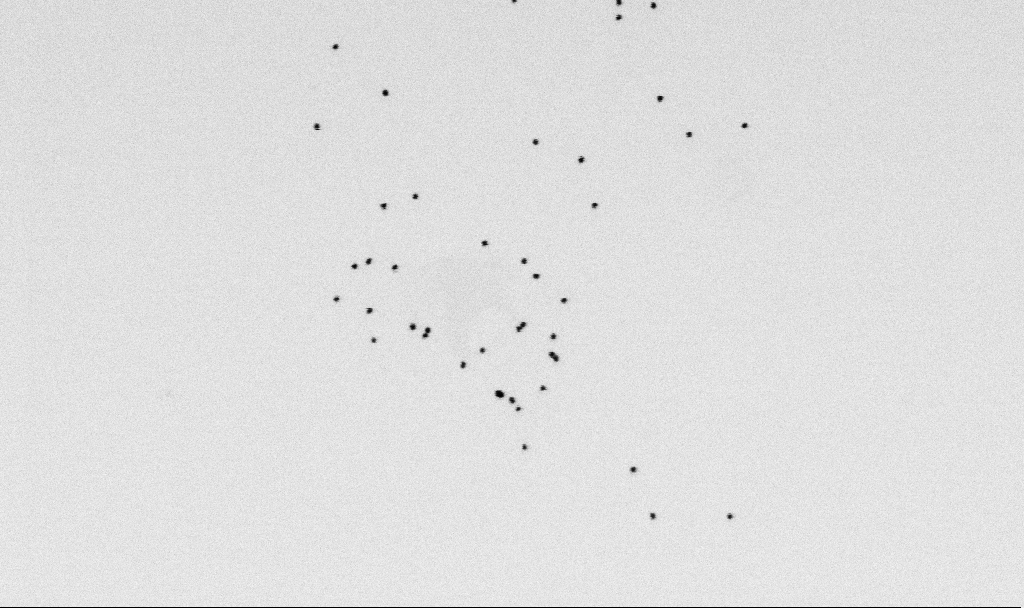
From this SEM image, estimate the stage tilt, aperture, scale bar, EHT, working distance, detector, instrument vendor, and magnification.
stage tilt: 0°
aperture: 30 µm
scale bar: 200 nm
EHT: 2 kV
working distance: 6.5 mm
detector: SE2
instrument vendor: Zeiss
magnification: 100 K X